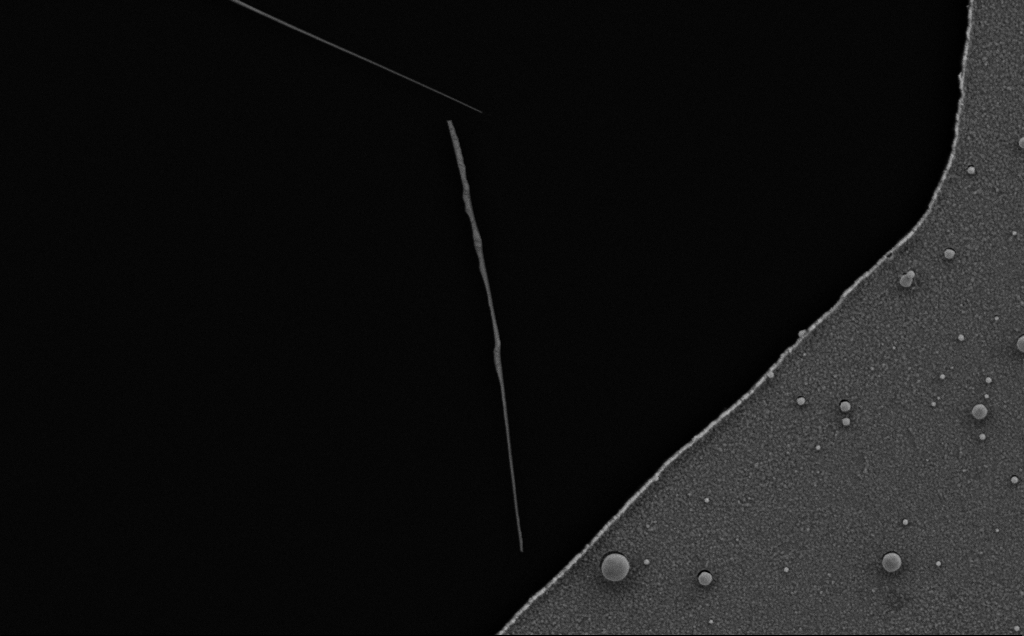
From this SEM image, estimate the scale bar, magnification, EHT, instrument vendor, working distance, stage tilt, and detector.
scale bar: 1000 nm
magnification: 29.73 K X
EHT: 5 kV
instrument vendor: Zeiss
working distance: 8 mm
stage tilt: -0.7°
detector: SE2